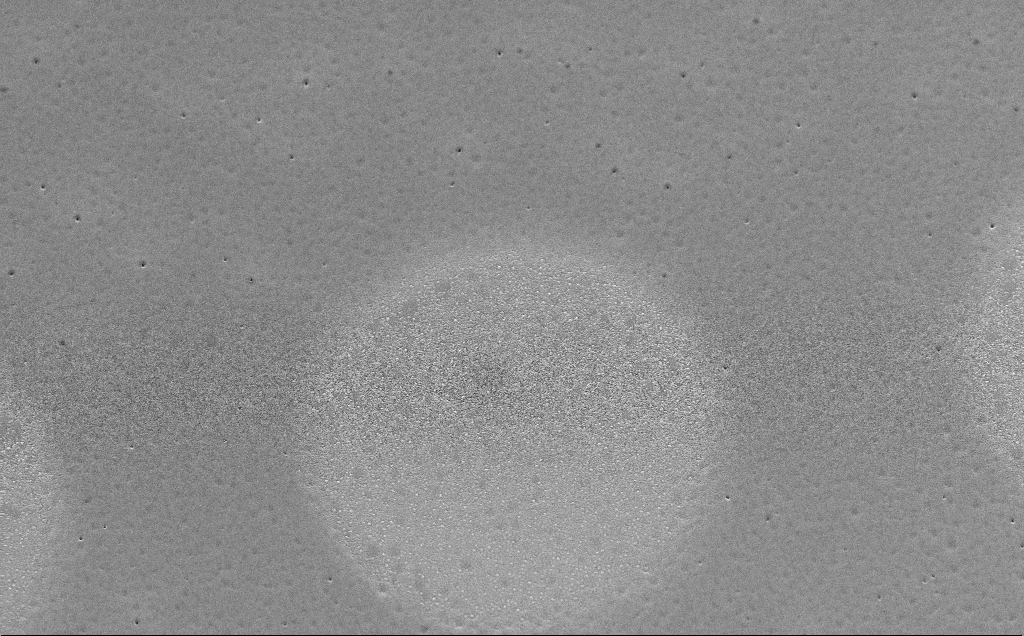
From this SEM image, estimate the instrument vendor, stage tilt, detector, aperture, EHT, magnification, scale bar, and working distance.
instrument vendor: Zeiss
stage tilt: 40.4°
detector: InLens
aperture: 30 µm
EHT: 10 kV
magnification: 7.94 K X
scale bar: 2000 nm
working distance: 6 mm